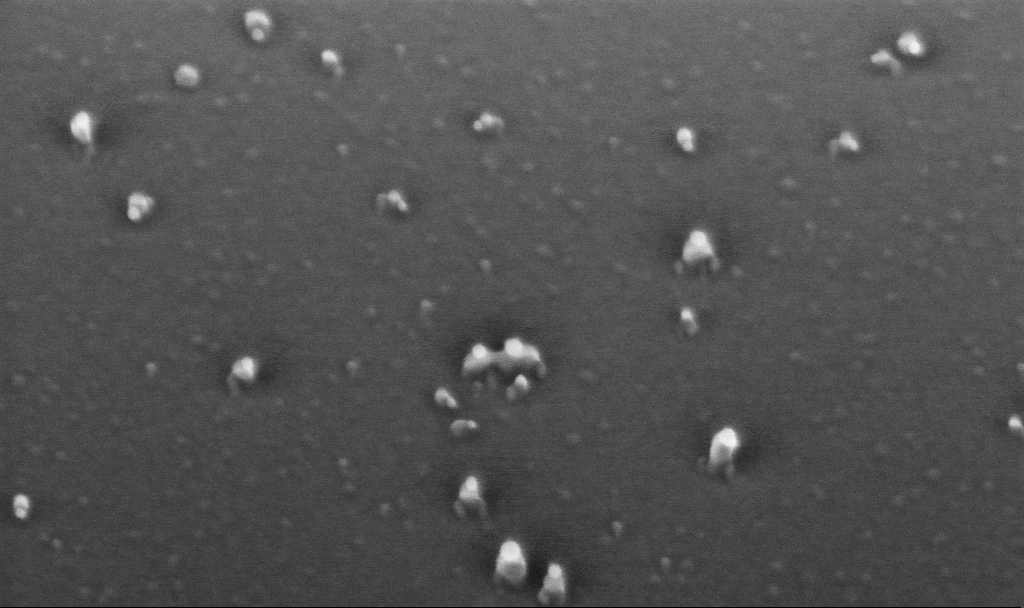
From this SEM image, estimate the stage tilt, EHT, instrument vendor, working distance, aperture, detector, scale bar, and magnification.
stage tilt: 45°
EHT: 10 kV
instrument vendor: Zeiss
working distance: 4.4 mm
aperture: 30 µm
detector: InLens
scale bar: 200 nm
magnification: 200 K X